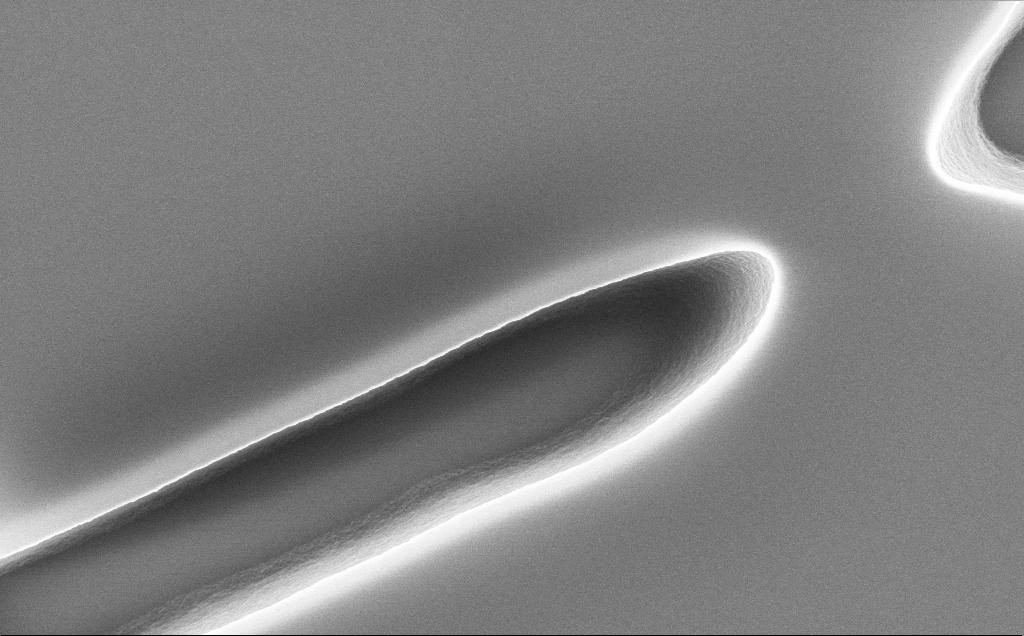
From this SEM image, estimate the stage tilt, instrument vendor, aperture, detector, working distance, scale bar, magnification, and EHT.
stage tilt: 0°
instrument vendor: Zeiss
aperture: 30 µm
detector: InLens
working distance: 12 mm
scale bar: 2000 nm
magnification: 36.1 K X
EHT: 10 kV